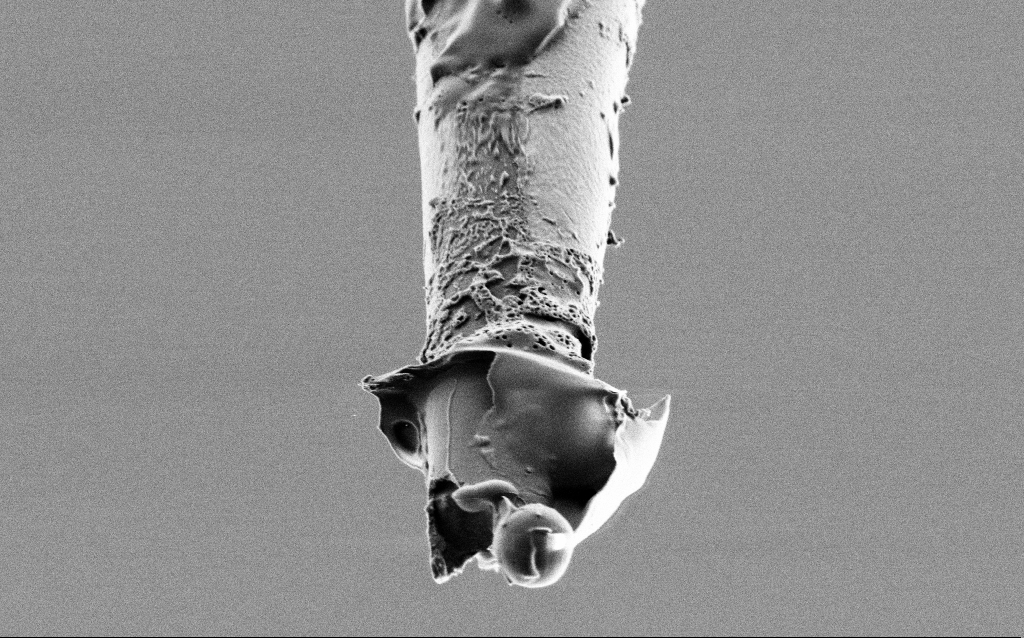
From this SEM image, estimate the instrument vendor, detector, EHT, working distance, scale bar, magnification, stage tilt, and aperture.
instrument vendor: Zeiss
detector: SE2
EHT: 1 kV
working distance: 6.5 mm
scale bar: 1000 nm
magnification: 25 K X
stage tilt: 45°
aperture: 30 µm